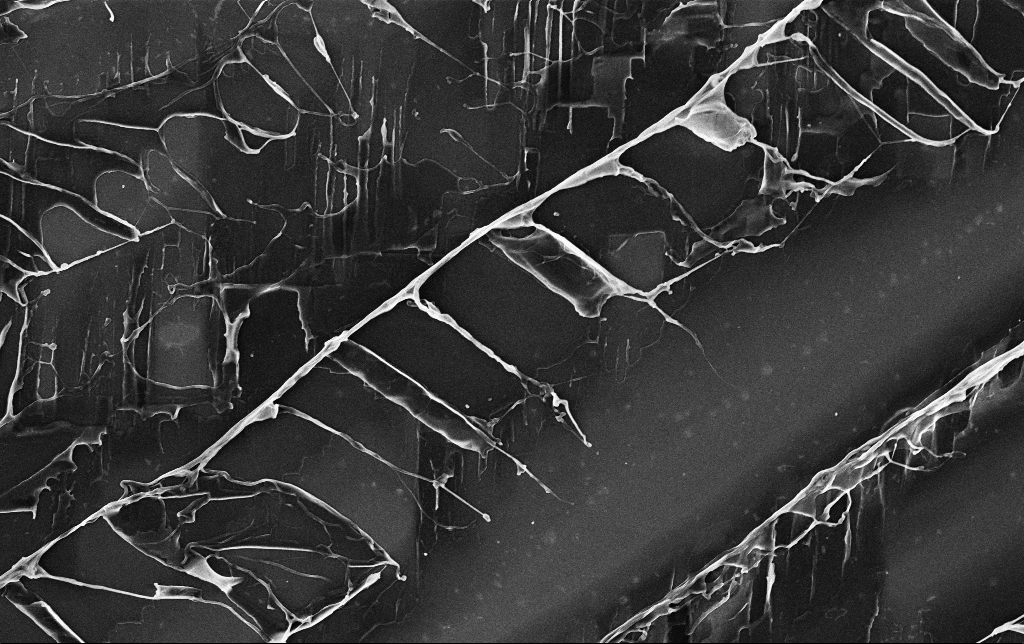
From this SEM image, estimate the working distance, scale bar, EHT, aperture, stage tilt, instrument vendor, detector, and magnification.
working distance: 3.5 mm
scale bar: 2000 nm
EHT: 3 kV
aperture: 30 µm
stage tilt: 0°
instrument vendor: Zeiss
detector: InLens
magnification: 7.95 K X